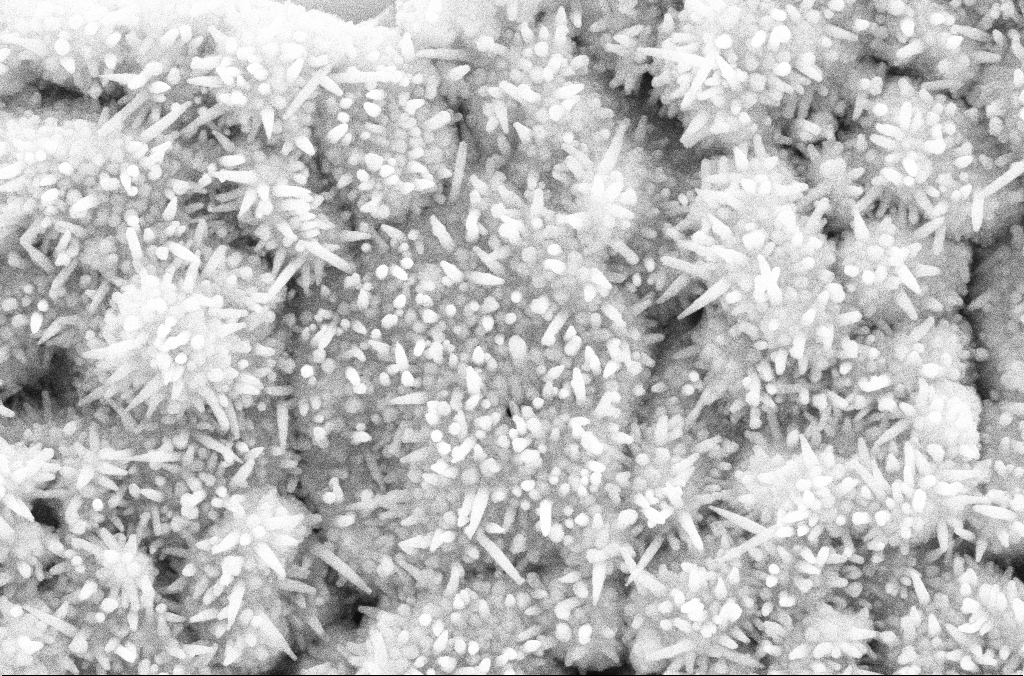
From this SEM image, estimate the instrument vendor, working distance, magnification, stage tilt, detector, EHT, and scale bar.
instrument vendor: Zeiss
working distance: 5.2 mm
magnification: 67 K X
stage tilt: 0.1°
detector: SE2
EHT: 10 kV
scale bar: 200 nm